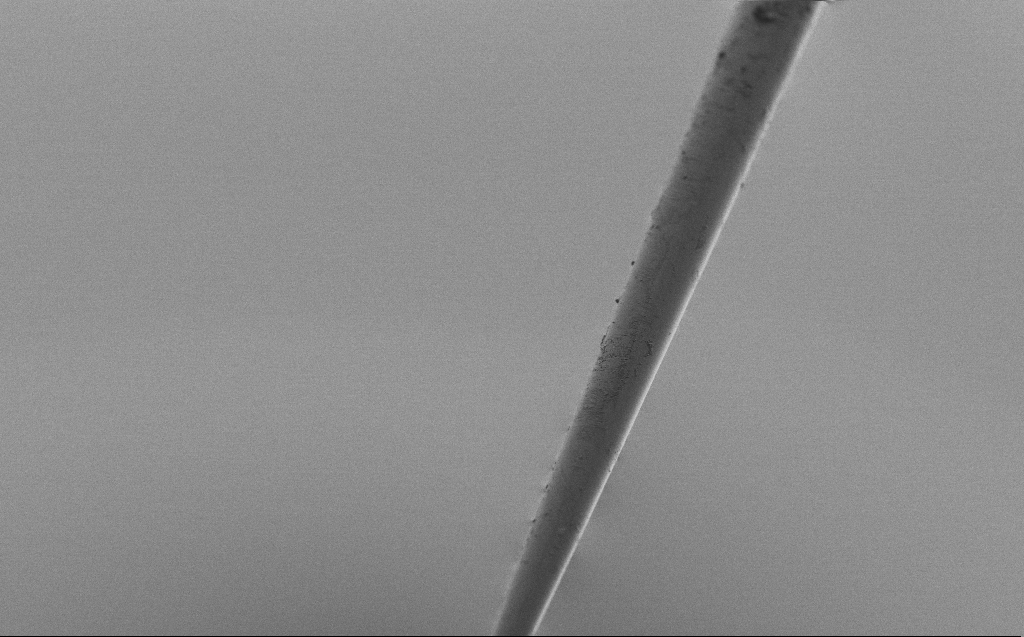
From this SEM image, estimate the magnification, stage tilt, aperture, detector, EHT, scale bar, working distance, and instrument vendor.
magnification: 1 K X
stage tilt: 45°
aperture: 30 µm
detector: SE2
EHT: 2 kV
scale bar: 20000 nm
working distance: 3 mm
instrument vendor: Zeiss